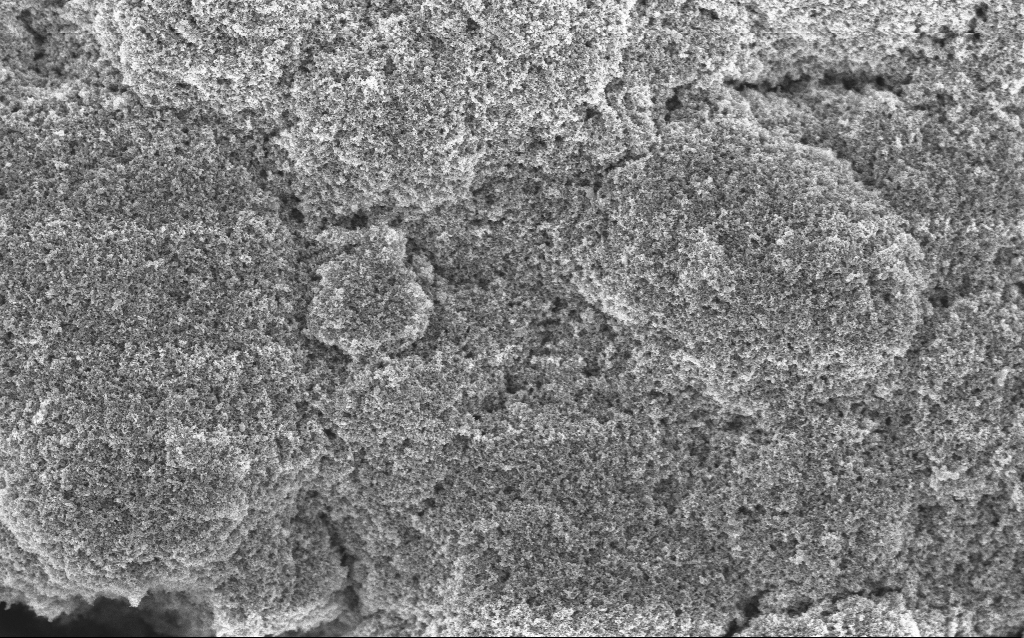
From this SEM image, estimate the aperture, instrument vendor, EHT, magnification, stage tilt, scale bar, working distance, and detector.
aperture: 30 µm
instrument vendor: Zeiss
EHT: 5 kV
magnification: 20.87 K X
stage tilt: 0°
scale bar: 1000 nm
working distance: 4.2 mm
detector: InLens